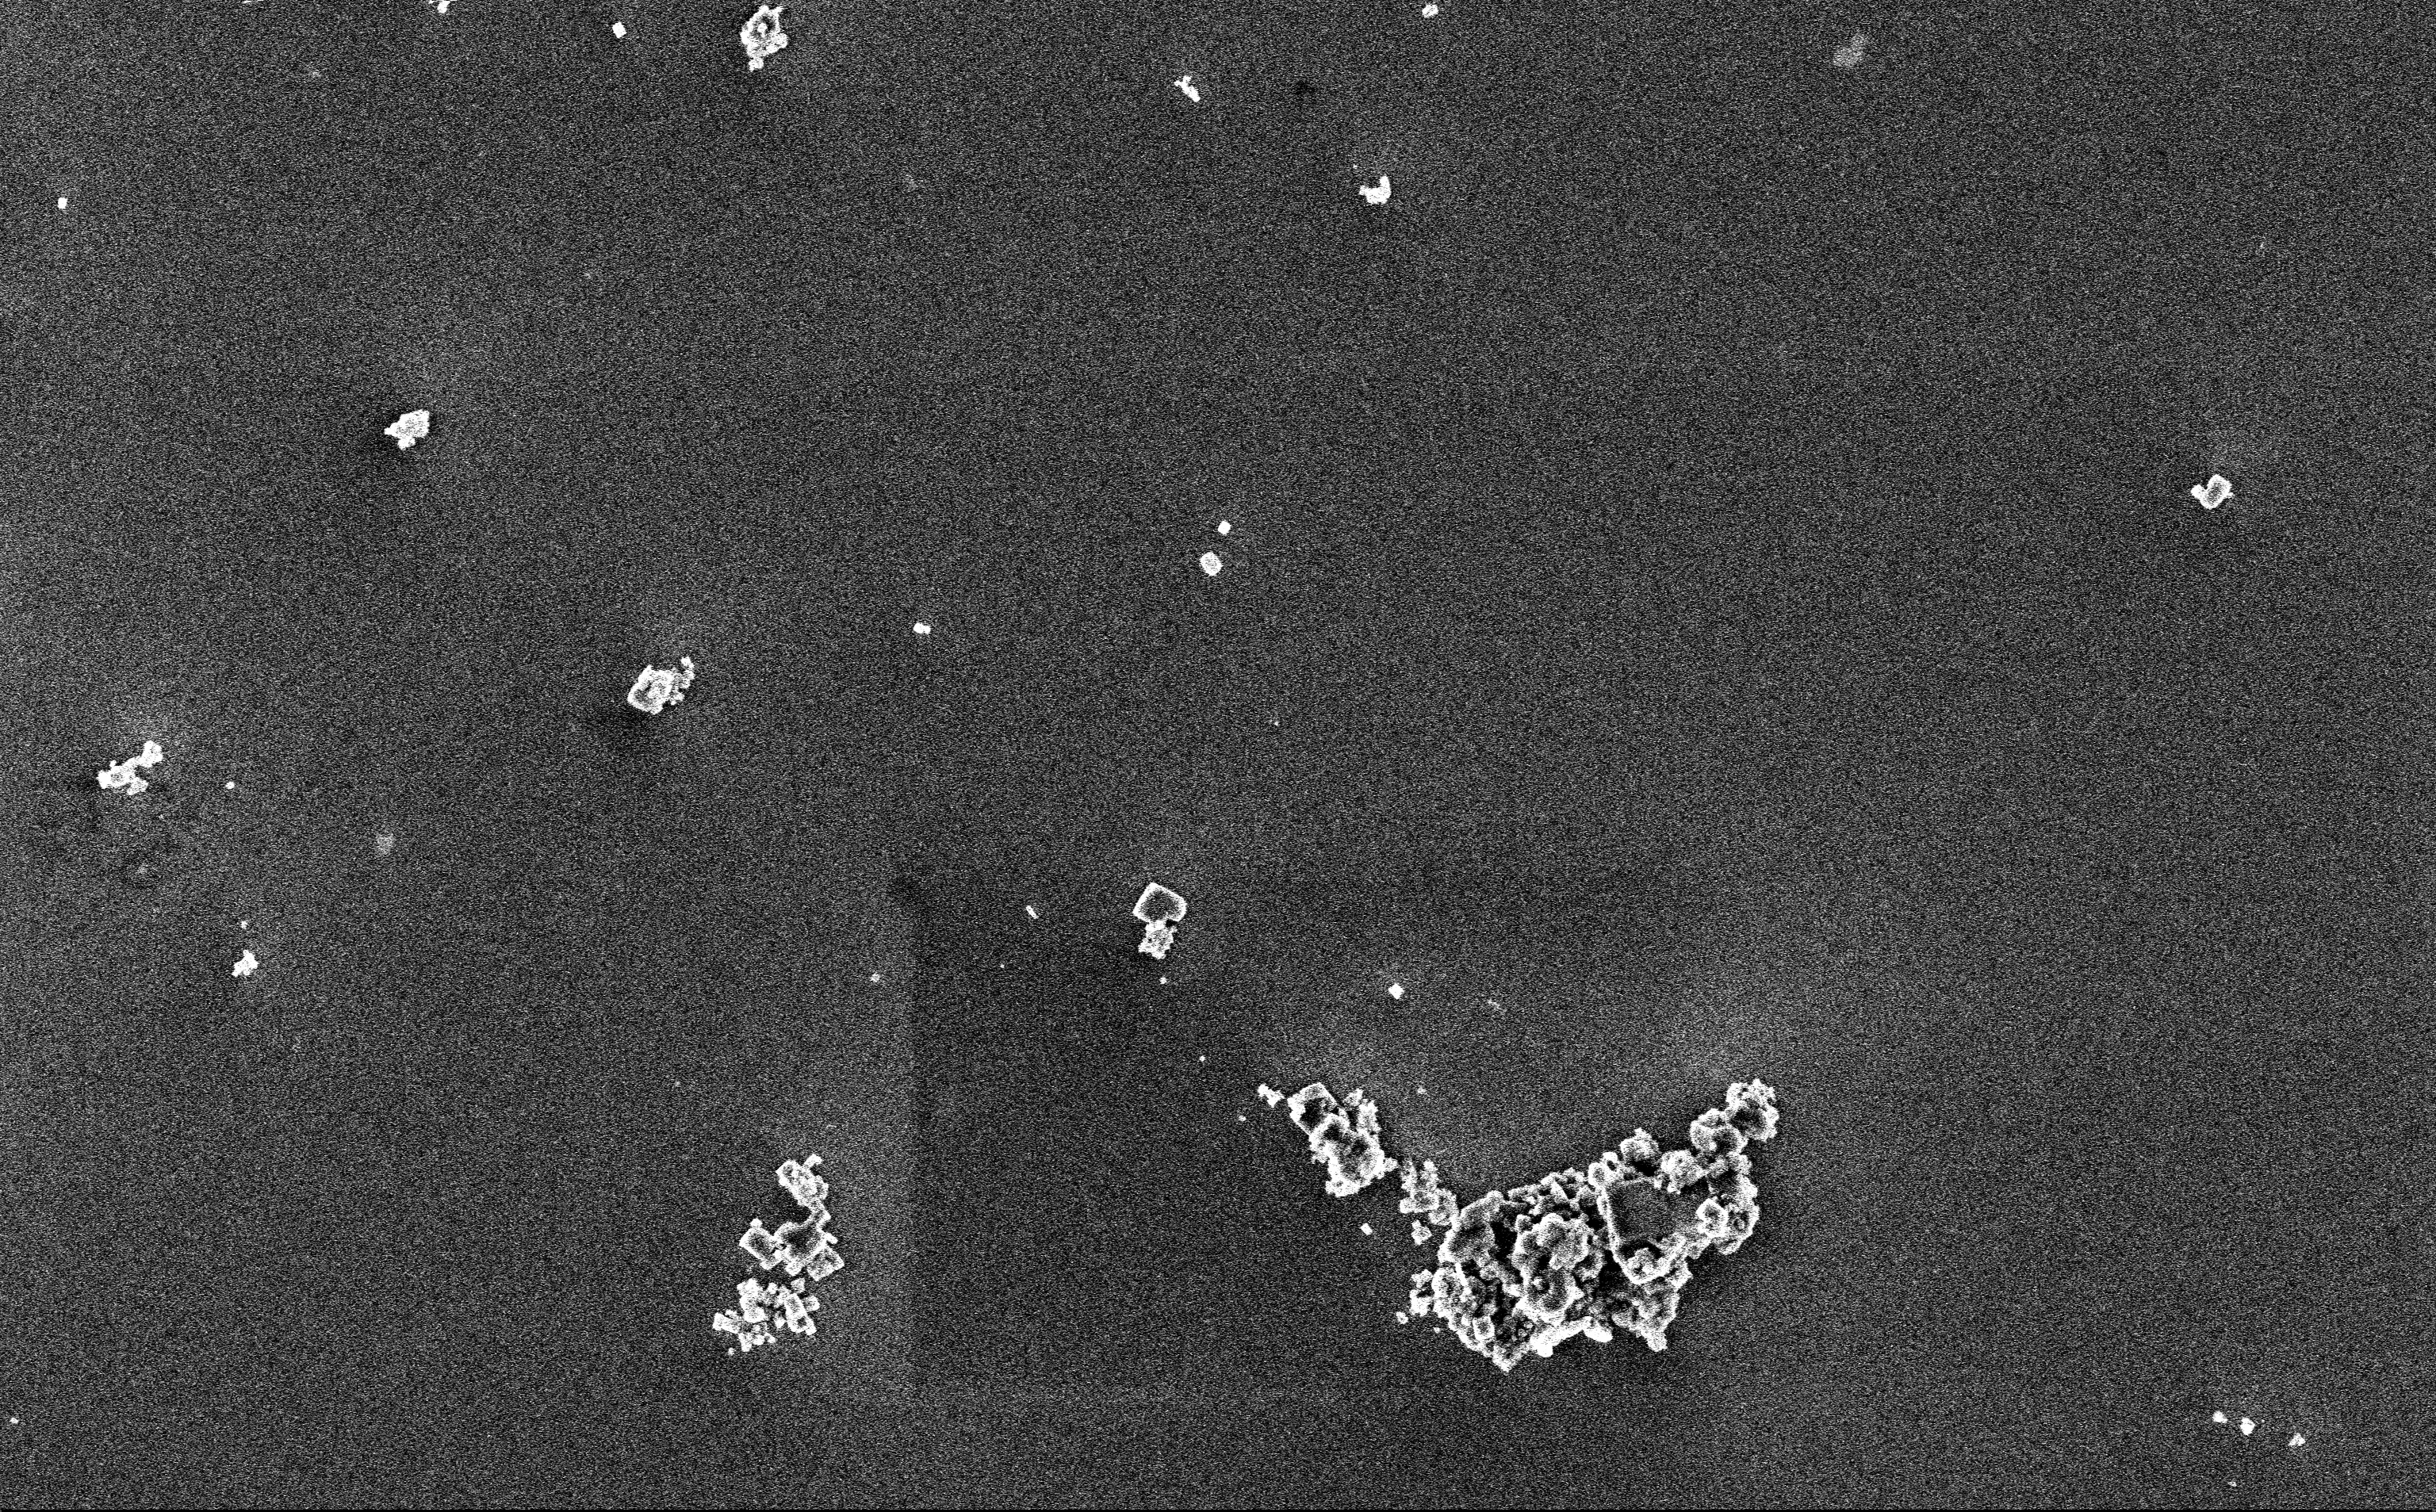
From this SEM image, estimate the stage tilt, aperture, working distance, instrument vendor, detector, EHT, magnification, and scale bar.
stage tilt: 0°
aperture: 30 µm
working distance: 3 mm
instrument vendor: Zeiss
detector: InLens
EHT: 3 kV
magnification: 12.85 K X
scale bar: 2000 nm